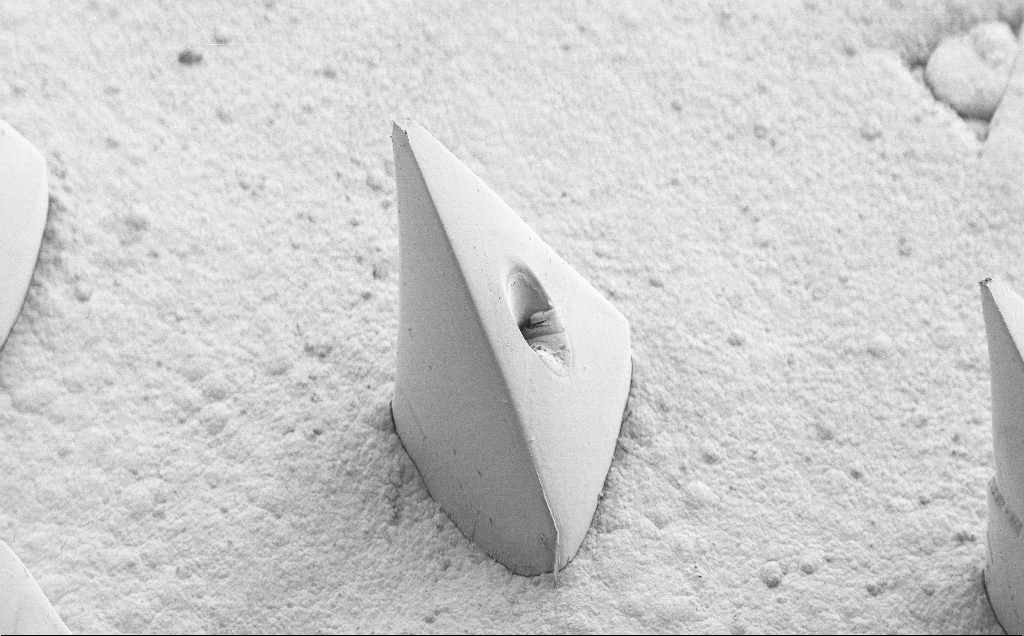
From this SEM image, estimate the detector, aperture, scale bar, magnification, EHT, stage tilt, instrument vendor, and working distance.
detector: SE2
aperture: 30 µm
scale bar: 100000 nm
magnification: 0.151 K X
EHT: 5 kV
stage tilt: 40°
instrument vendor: Zeiss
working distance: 9 mm